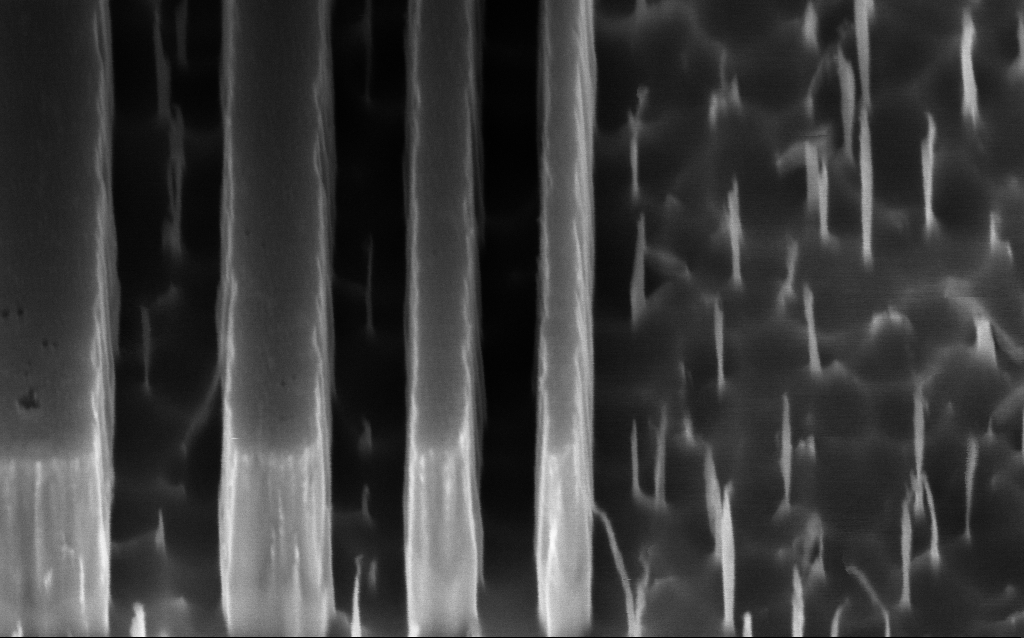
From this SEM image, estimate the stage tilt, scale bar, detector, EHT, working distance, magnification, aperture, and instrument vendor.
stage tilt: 45°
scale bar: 200 nm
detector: InLens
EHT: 3 kV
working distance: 5 mm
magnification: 136.57 K X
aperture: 30 µm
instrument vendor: Zeiss